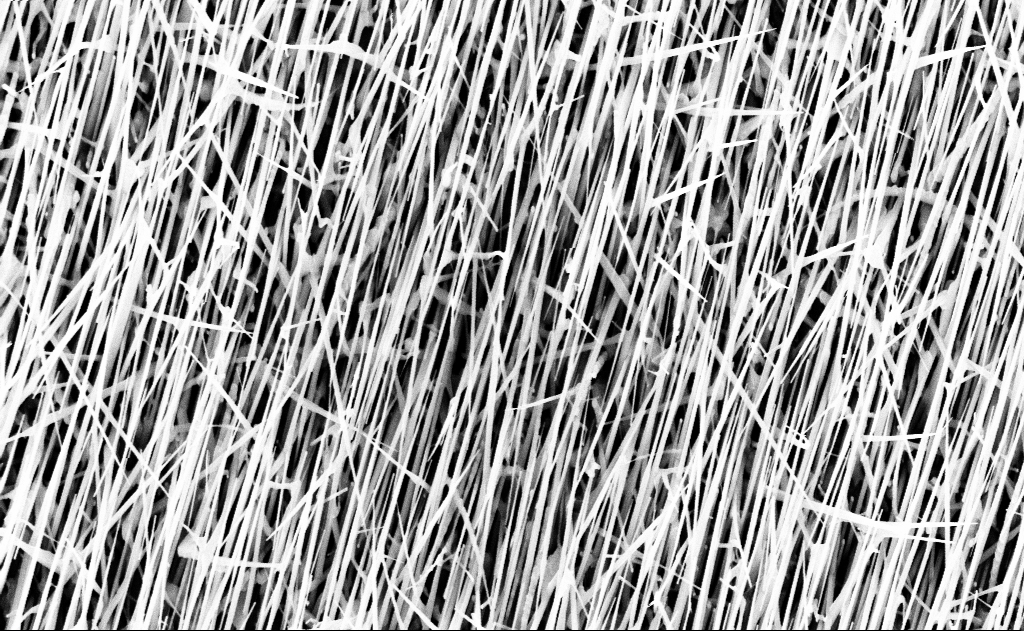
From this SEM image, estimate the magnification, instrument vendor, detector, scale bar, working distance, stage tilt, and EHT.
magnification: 20 K X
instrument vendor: Zeiss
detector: InLens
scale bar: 1000 nm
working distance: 16 mm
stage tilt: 0°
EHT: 10 kV